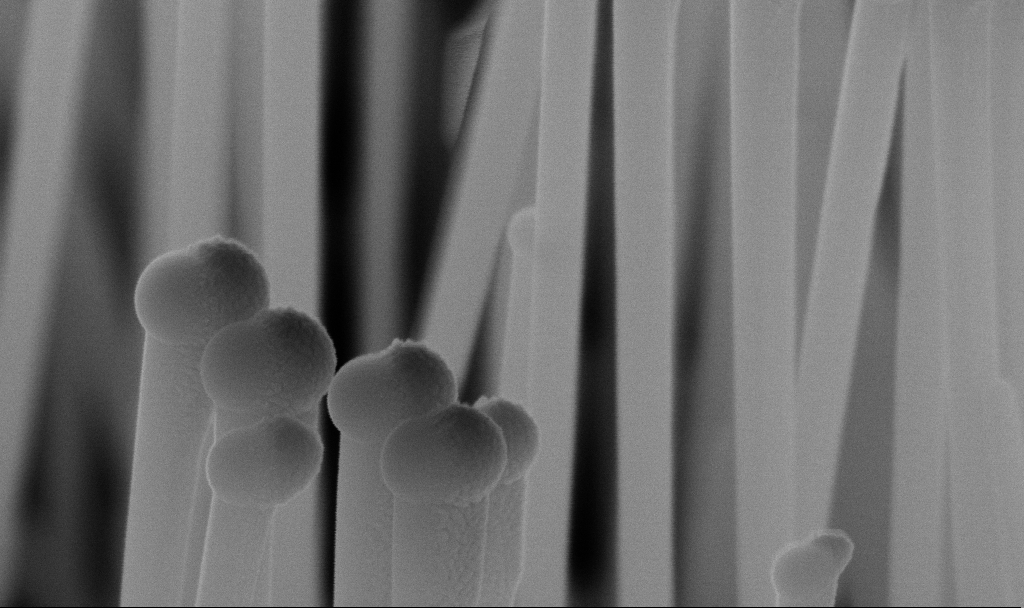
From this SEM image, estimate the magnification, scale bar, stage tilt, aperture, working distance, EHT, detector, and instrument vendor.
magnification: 181.5 K X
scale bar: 200 nm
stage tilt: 45°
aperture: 30 µm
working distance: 7.2 mm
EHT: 10 kV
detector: InLens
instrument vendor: Zeiss